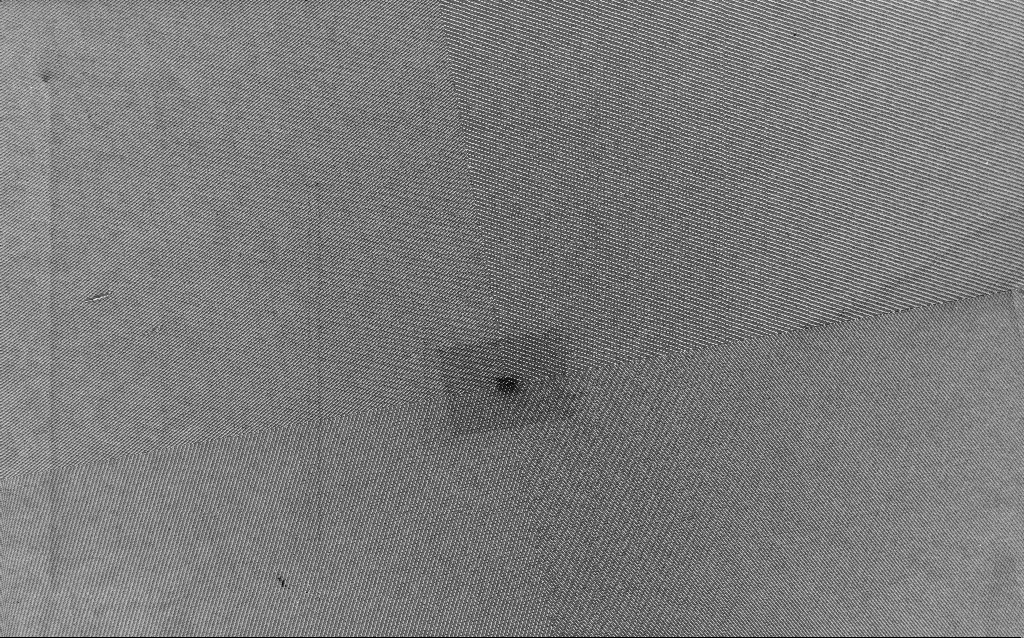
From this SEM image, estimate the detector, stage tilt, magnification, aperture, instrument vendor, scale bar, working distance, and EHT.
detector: InLens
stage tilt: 0°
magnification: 1.41 K X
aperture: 30 µm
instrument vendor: Zeiss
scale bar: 10000 nm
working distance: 4.5 mm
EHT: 3 kV